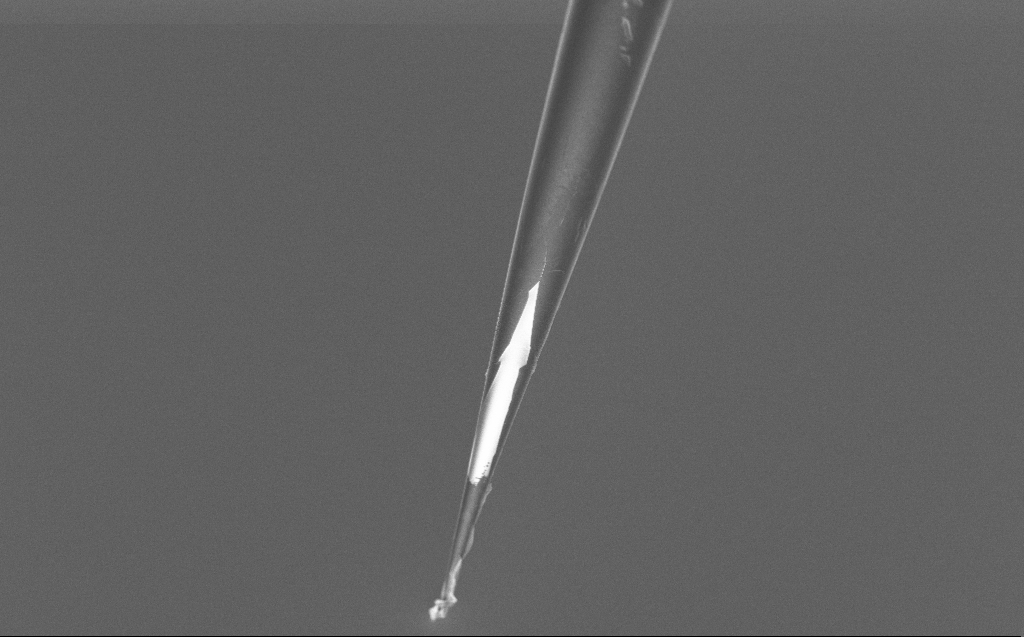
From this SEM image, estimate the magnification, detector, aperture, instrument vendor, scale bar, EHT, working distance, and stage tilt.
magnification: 2.5 K X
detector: InLens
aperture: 30 µm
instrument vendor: Zeiss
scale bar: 10000 nm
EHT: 5 kV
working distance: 6 mm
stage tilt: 45°